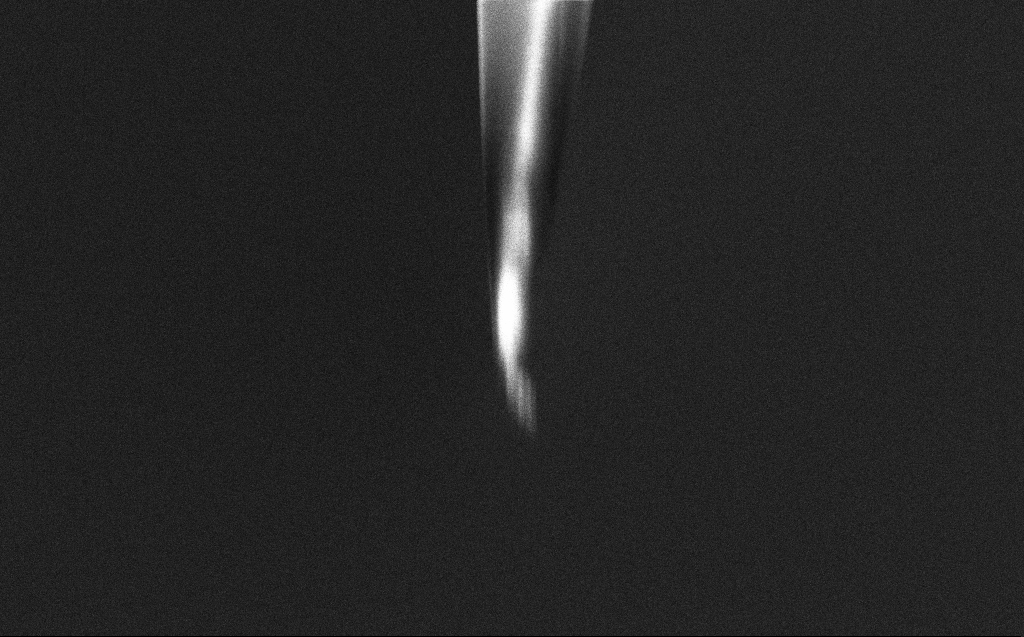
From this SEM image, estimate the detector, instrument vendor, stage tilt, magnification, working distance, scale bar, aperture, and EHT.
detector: InLens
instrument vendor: Zeiss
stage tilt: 45°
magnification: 13.42 K X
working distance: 5 mm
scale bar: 2000 nm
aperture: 20 µm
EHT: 2 kV